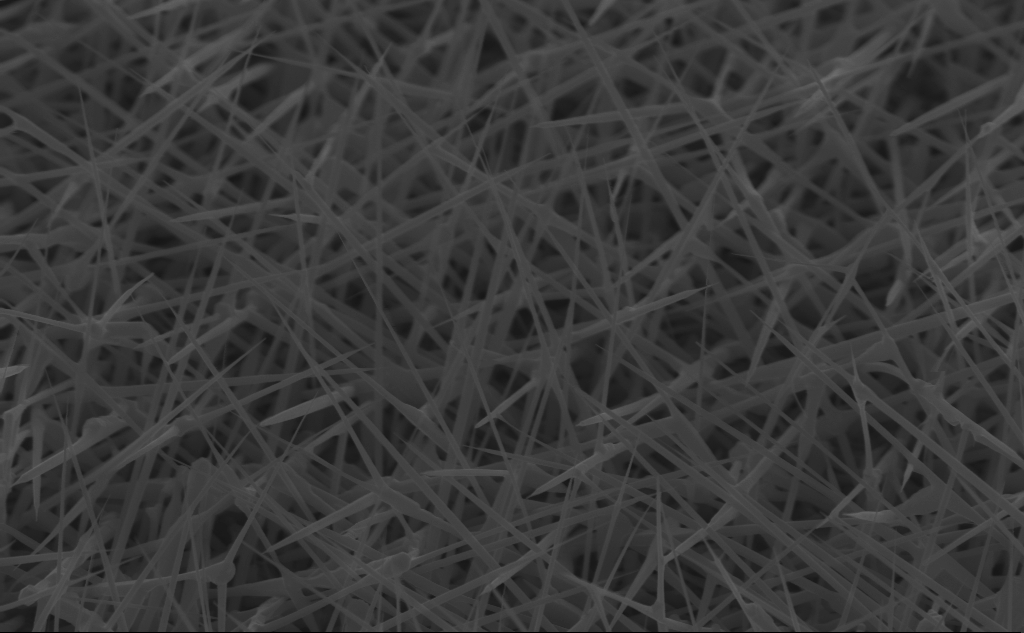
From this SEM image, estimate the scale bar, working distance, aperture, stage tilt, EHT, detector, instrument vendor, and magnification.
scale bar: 1000 nm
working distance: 5 mm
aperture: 30 µm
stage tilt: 45°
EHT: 10 kV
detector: InLens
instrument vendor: Zeiss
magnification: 40 K X